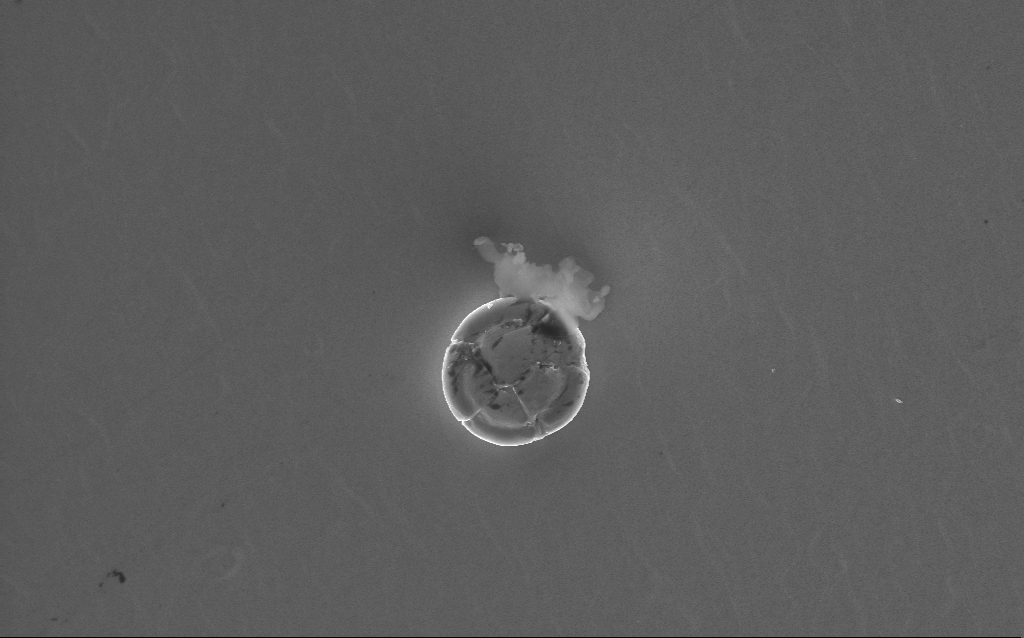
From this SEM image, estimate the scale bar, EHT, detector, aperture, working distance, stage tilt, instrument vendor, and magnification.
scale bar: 2000 nm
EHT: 10 kV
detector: InLens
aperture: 30 µm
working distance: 2 mm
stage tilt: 0°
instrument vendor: Zeiss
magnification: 13.42 K X